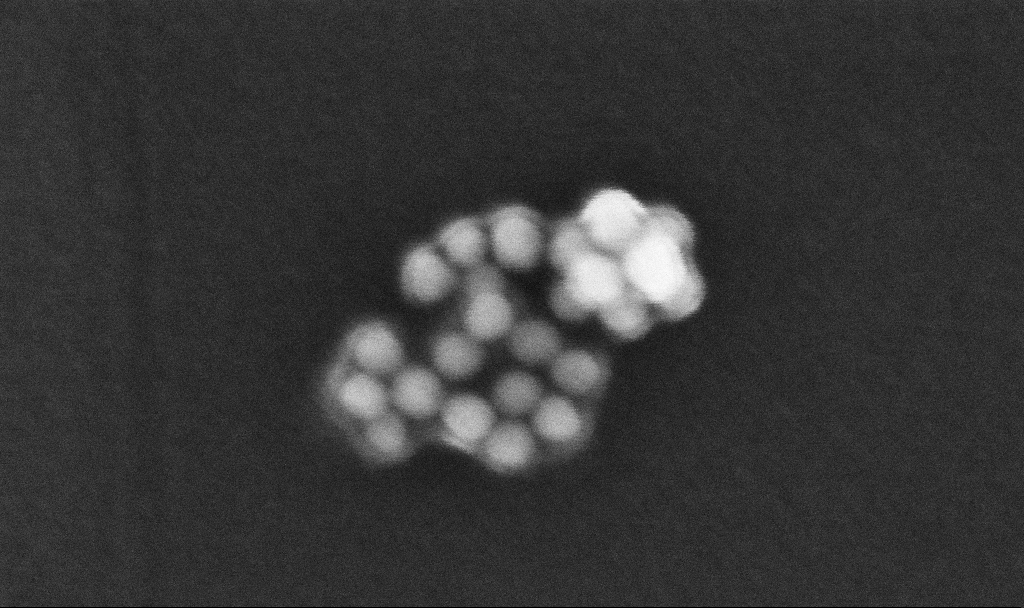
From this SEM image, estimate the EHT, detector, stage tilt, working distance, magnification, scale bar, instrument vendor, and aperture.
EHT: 10 kV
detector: InLens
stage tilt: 0°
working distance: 3.4 mm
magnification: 891.23 K X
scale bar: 20 nm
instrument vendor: Zeiss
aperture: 30 µm